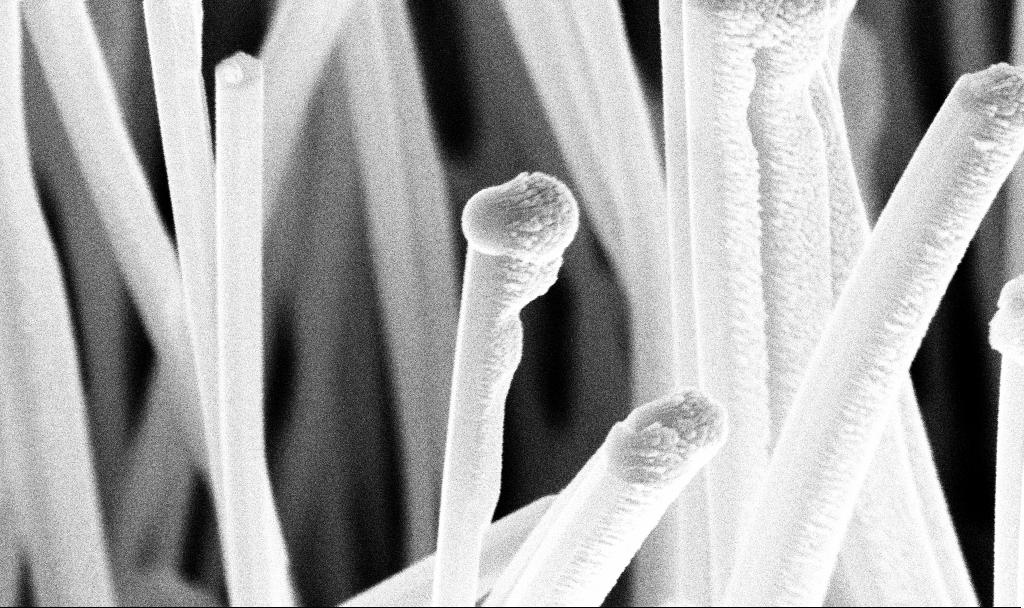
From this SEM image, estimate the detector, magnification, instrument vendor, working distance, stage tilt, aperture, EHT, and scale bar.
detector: InLens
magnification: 147.64 K X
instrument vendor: Zeiss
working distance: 7.2 mm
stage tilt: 45°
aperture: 30 µm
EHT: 10 kV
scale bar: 200 nm